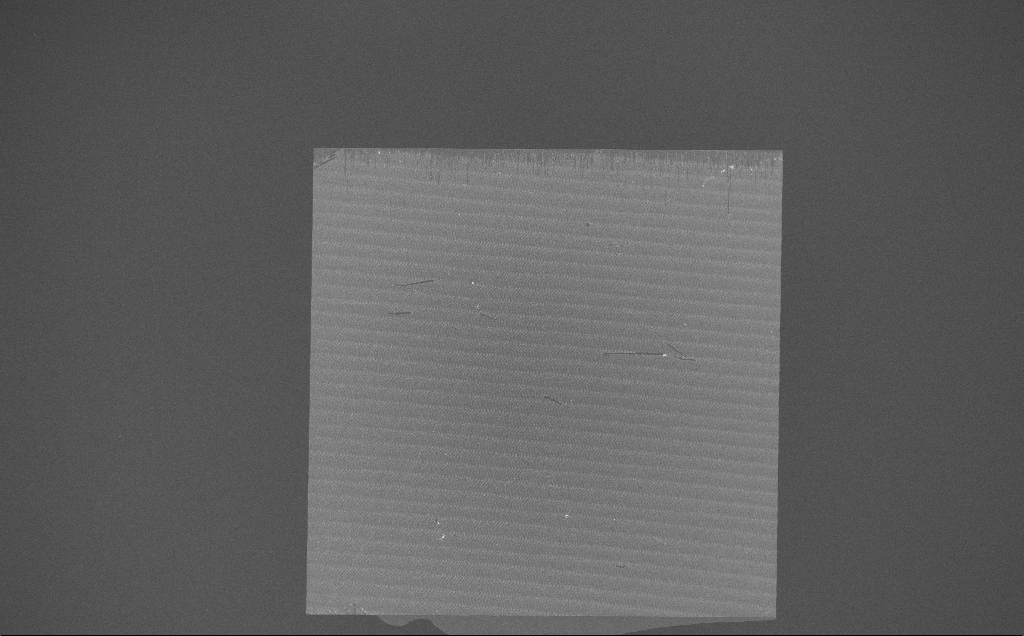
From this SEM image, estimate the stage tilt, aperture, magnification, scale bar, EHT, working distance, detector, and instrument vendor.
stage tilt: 0°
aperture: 30 µm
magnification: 0.174 K X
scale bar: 100000 nm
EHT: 10 kV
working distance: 7 mm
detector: InLens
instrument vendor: Zeiss